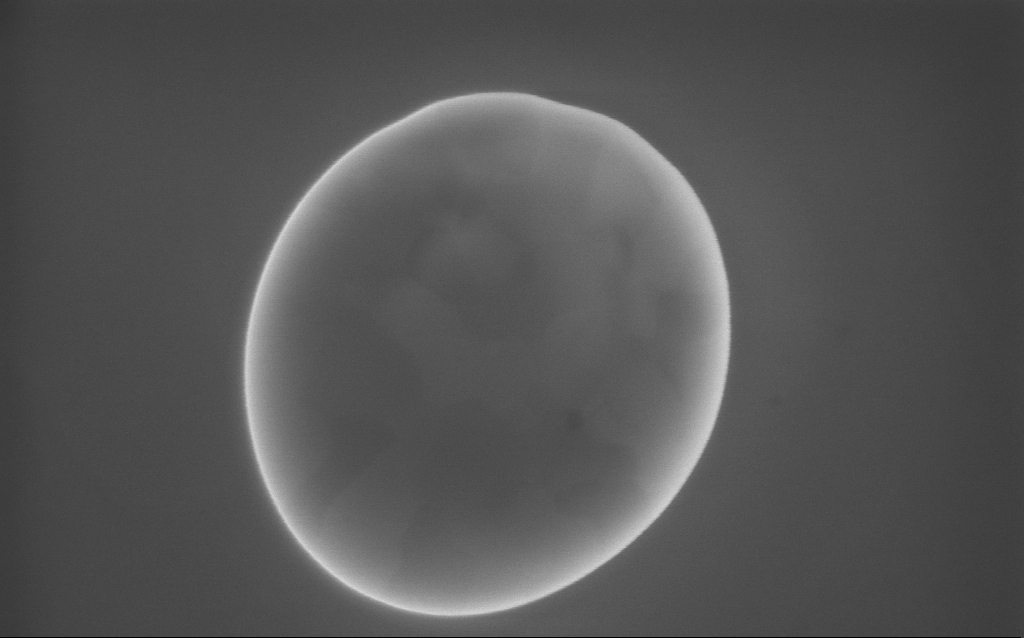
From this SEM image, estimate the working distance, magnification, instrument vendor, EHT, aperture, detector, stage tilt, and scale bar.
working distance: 2 mm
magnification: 97.47 K X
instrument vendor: Zeiss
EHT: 10 kV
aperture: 30 µm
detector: InLens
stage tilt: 0°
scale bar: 200 nm